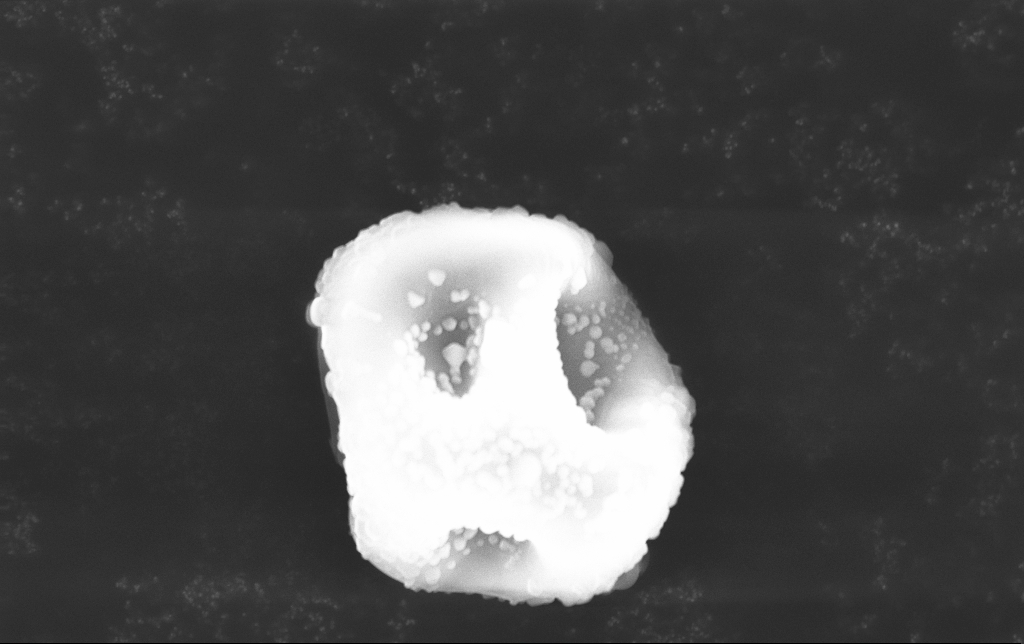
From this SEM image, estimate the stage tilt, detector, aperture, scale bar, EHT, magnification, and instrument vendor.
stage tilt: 0°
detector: InLens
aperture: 30 µm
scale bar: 2000 nm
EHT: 15 kV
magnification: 12.46 K X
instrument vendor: Zeiss